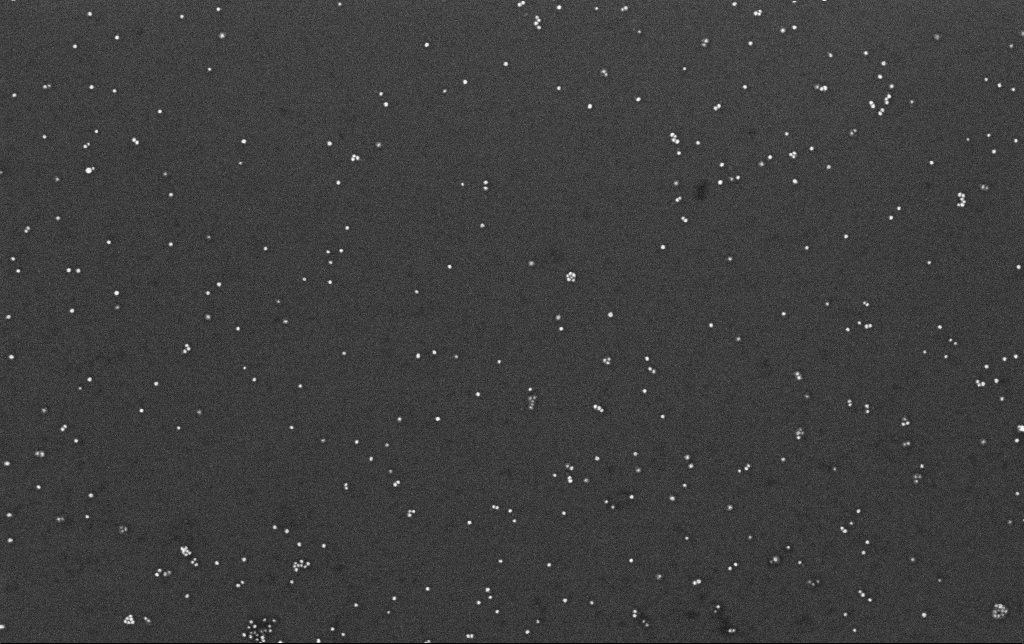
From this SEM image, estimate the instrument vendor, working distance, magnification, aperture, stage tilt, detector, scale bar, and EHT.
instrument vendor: Zeiss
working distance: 3.4 mm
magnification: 100 K X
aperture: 30 µm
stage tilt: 0°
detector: InLens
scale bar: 200 nm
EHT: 10 kV